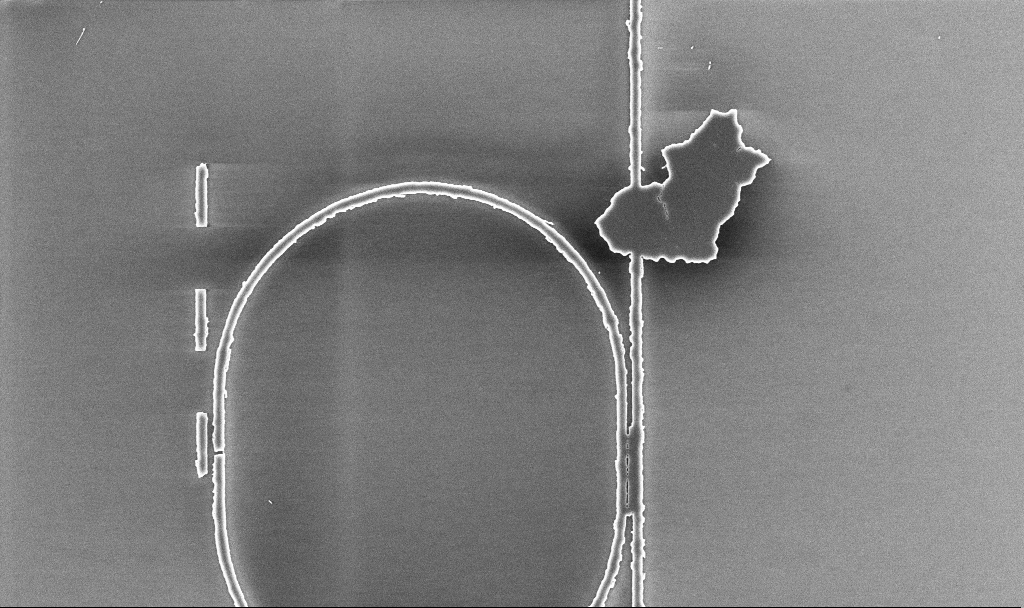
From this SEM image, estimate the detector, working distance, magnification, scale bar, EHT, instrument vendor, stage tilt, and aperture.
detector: InLens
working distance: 5.2 mm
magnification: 7.67 K X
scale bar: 2000 nm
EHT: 5 kV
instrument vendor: Zeiss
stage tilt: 0°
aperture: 30 µm